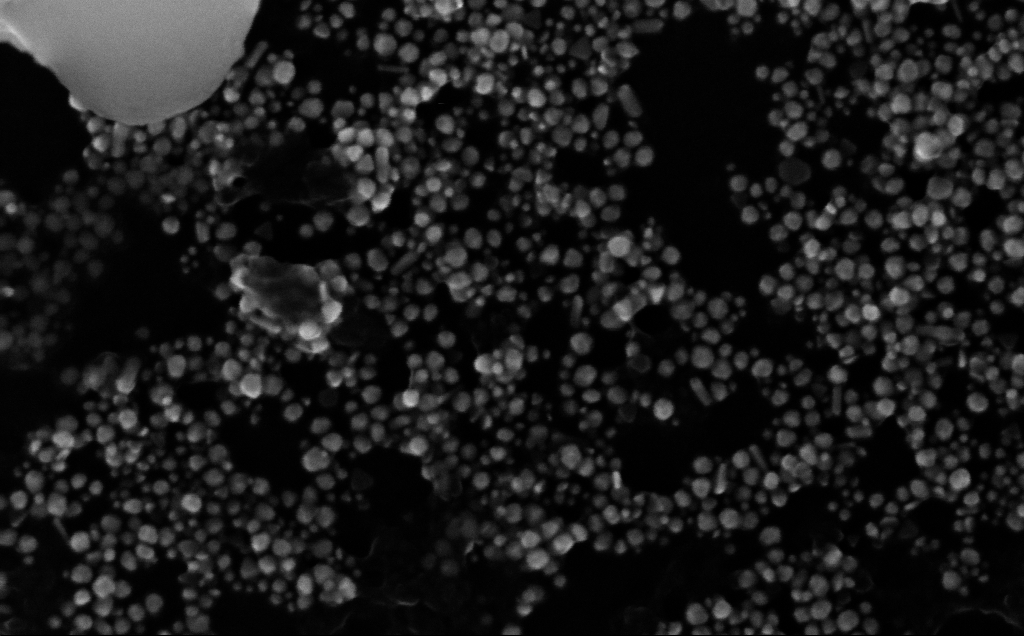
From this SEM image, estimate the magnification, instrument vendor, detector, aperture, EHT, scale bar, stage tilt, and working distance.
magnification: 307.55 K X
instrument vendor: Zeiss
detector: InLens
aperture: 30 µm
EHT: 10 kV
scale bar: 100 nm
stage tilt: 0°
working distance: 4 mm